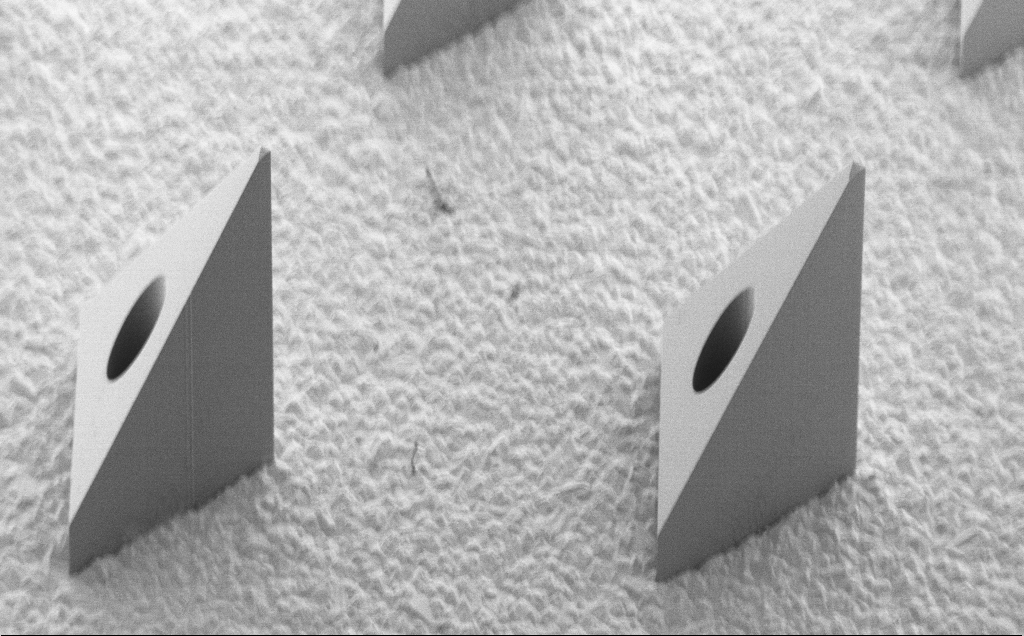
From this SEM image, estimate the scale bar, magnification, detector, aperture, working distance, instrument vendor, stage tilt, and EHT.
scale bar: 200000 nm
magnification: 0.139 K X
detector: SE2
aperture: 30 µm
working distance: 8 mm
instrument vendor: Zeiss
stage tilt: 40°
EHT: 5 kV